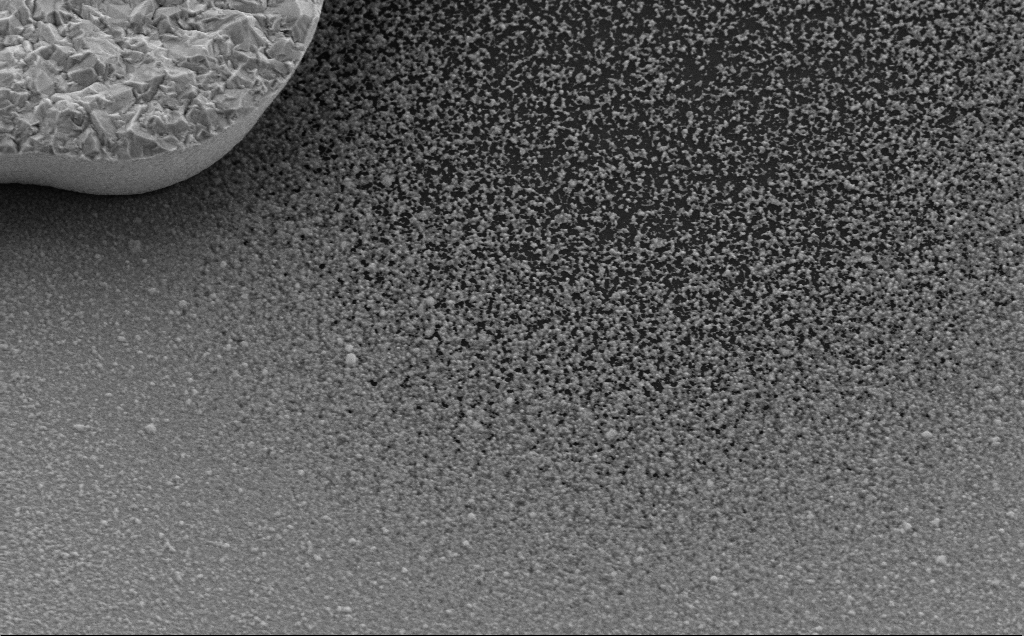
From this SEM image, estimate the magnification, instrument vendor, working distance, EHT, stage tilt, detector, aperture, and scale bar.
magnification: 26.27 K X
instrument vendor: Zeiss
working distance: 9 mm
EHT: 5 kV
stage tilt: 30°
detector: SE2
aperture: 30 µm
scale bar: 1000 nm